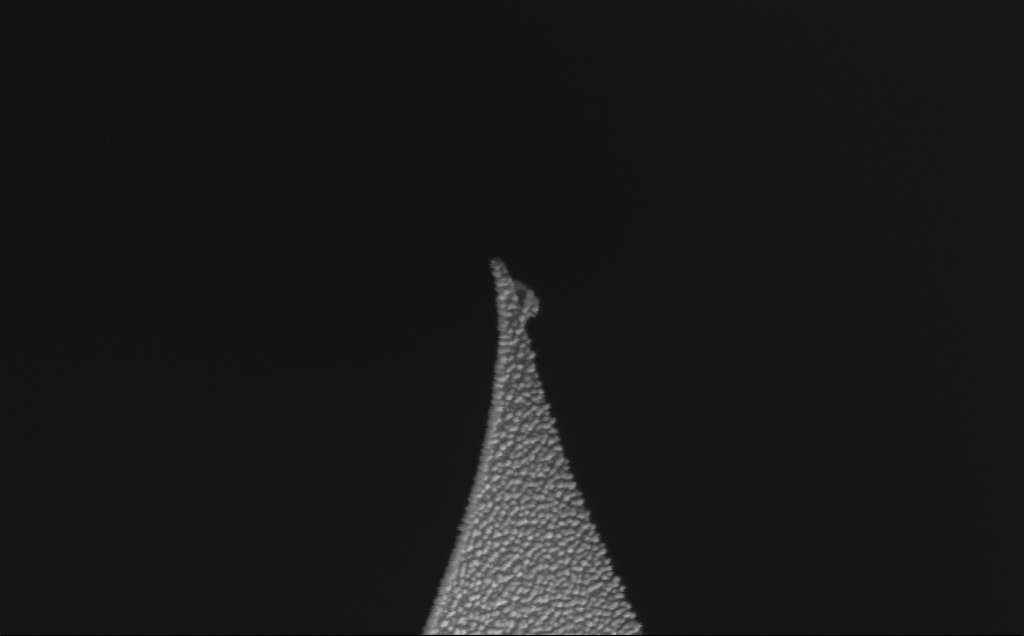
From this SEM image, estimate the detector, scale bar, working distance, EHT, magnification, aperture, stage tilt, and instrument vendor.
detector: InLens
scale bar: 100 nm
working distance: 8 mm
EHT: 10 kV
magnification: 233.28 K X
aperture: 30 µm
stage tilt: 52.7°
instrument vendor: Zeiss